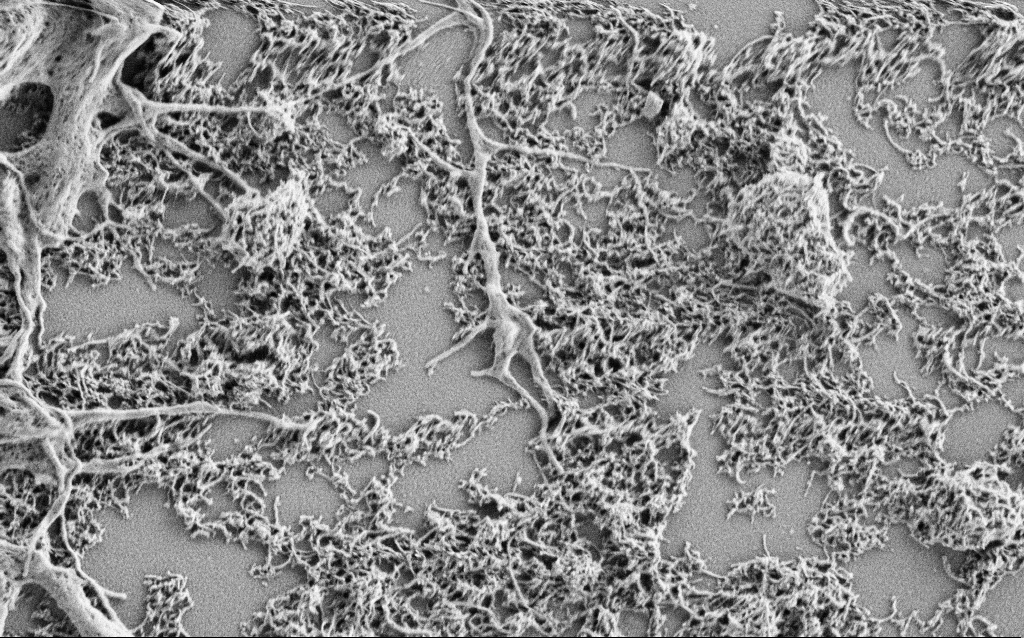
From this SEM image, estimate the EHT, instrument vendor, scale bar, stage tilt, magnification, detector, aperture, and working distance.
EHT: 1 kV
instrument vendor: Zeiss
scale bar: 1000 nm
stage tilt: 0°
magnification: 20 K X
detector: SE2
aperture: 30 µm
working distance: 3 mm